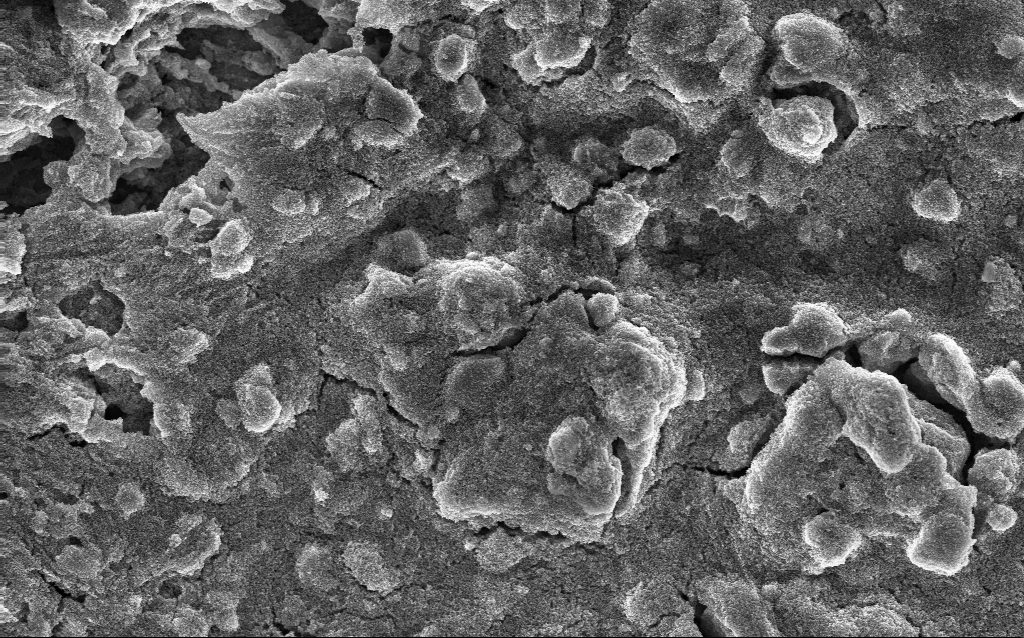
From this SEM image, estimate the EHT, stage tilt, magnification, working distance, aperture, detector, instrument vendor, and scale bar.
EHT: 10 kV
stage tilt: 0°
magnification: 5.83 K X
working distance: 2.6 mm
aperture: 30 µm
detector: InLens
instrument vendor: Zeiss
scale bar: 10000 nm